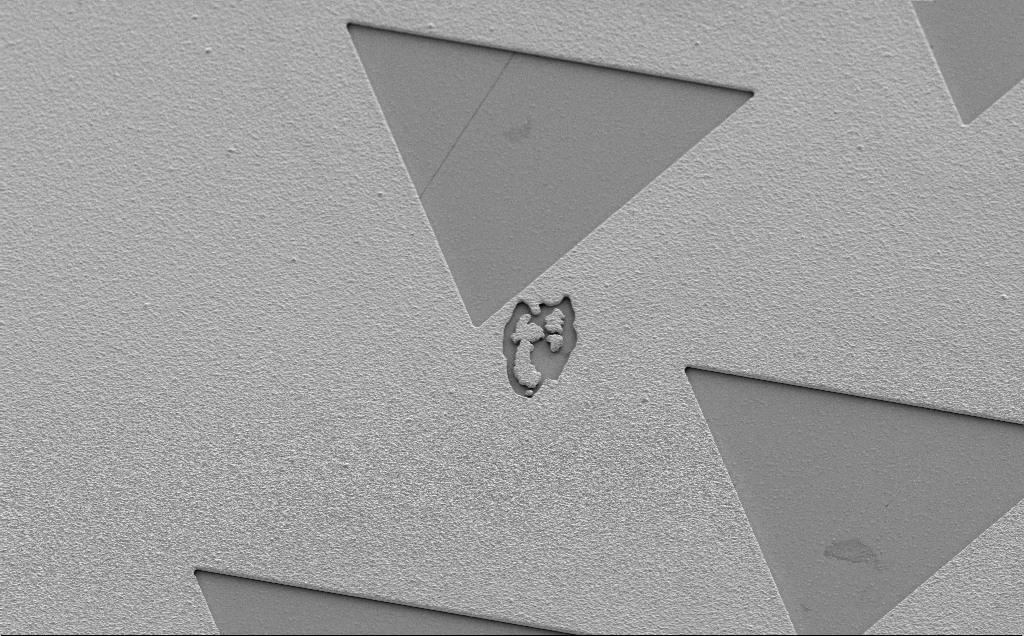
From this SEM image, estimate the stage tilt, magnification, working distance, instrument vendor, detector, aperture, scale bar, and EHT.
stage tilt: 35°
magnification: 1.33 K X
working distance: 13 mm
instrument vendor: Zeiss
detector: SE2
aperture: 30 µm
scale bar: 20000 nm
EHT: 5 kV